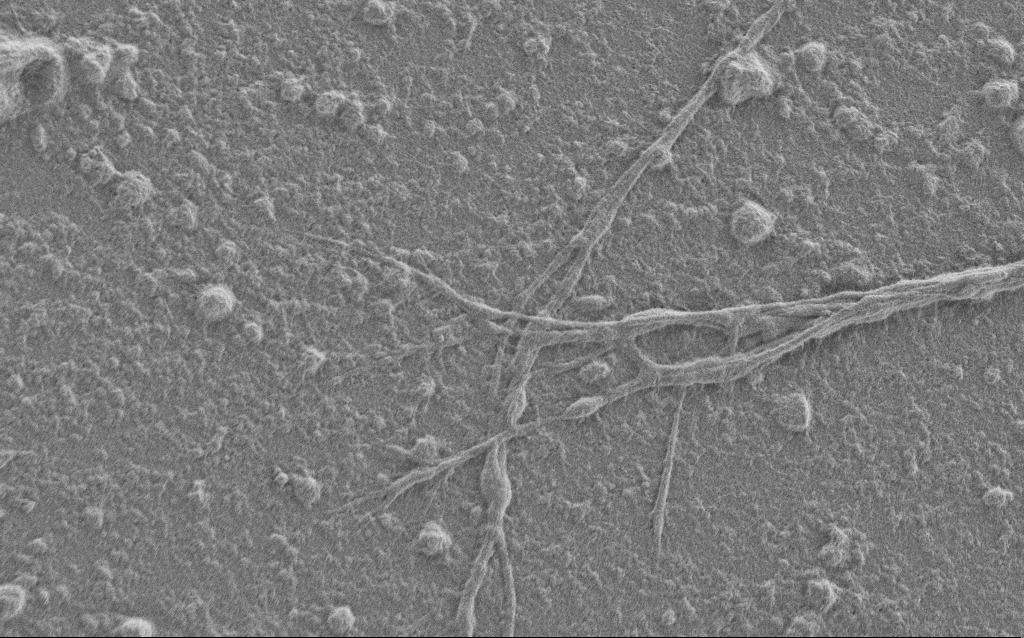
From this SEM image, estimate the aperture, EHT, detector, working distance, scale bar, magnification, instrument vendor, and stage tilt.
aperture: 30 µm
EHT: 1 kV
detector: SE2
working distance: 6 mm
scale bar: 10000 nm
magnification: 6 K X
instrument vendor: Zeiss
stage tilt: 0°